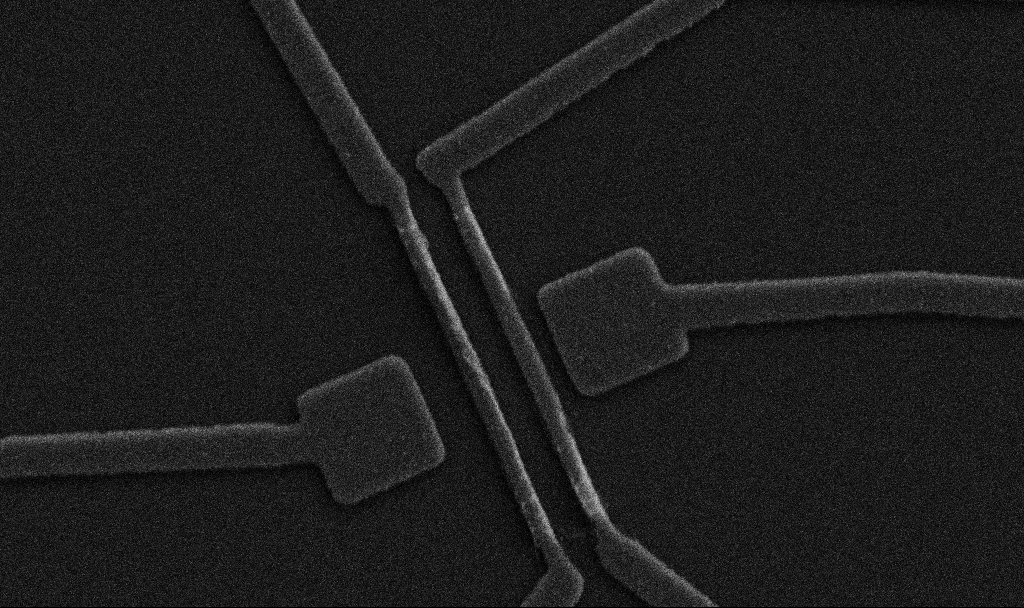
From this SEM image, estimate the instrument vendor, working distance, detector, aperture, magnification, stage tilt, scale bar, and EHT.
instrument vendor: Zeiss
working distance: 10.7 mm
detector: SE2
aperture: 30 µm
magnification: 20 K X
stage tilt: -0°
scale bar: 1000 nm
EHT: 5 kV